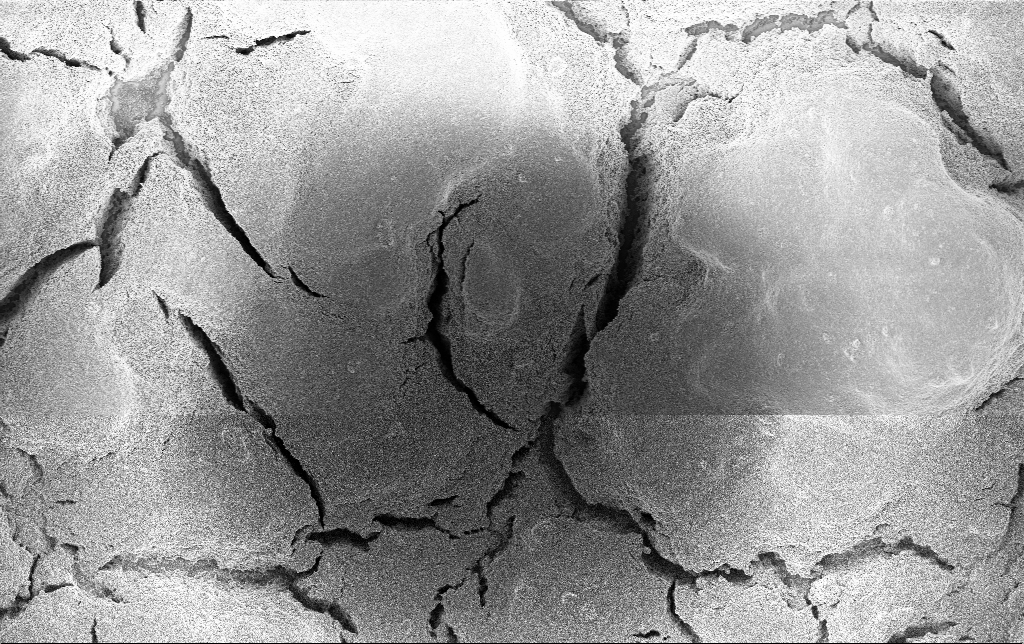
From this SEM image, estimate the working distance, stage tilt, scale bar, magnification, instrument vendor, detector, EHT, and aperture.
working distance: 2.8 mm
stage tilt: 0°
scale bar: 10000 nm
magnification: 1.5 K X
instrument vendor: Zeiss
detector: InLens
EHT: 3 kV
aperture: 30 µm